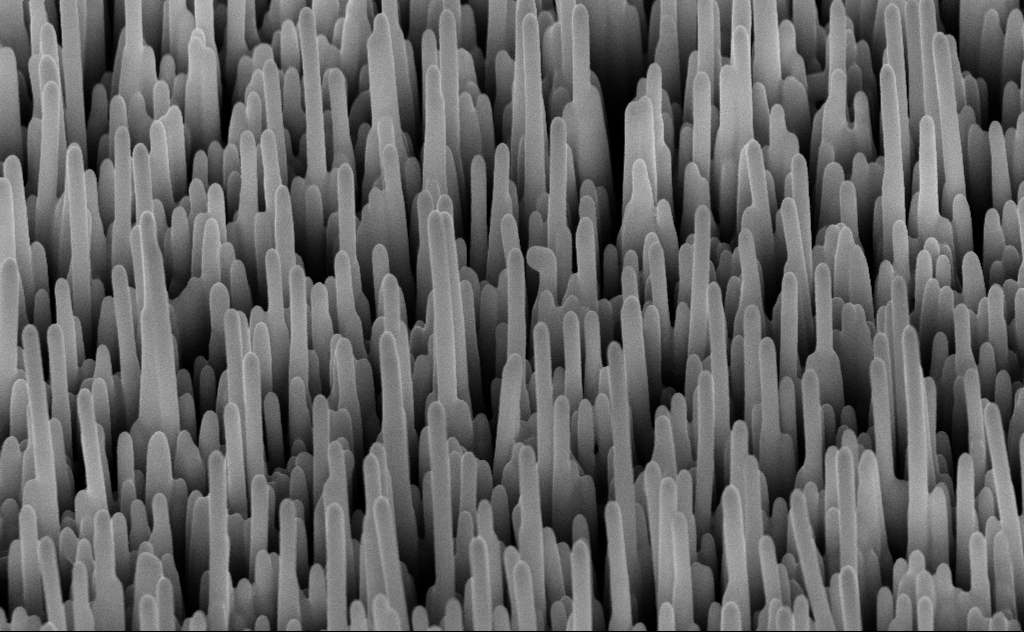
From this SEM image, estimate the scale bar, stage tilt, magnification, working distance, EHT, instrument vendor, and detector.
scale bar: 1000 nm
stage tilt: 45°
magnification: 40 K X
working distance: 8 mm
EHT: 10 kV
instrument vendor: Zeiss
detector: InLens